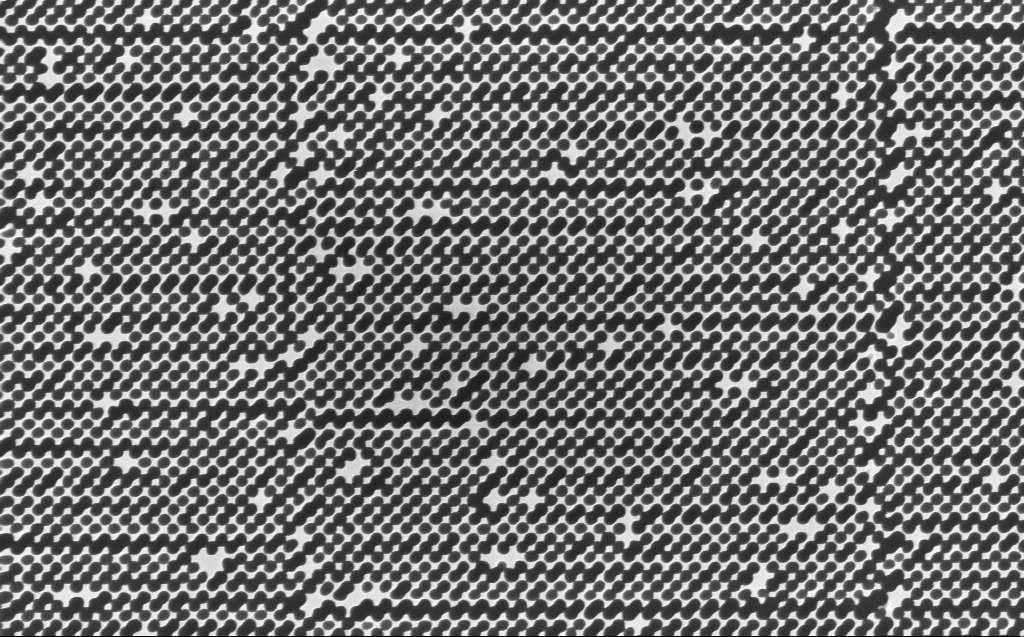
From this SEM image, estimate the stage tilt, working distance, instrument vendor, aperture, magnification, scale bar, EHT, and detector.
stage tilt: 0°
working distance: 7 mm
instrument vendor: Zeiss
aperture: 30 µm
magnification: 47.29 K X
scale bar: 1000 nm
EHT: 5 kV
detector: InLens